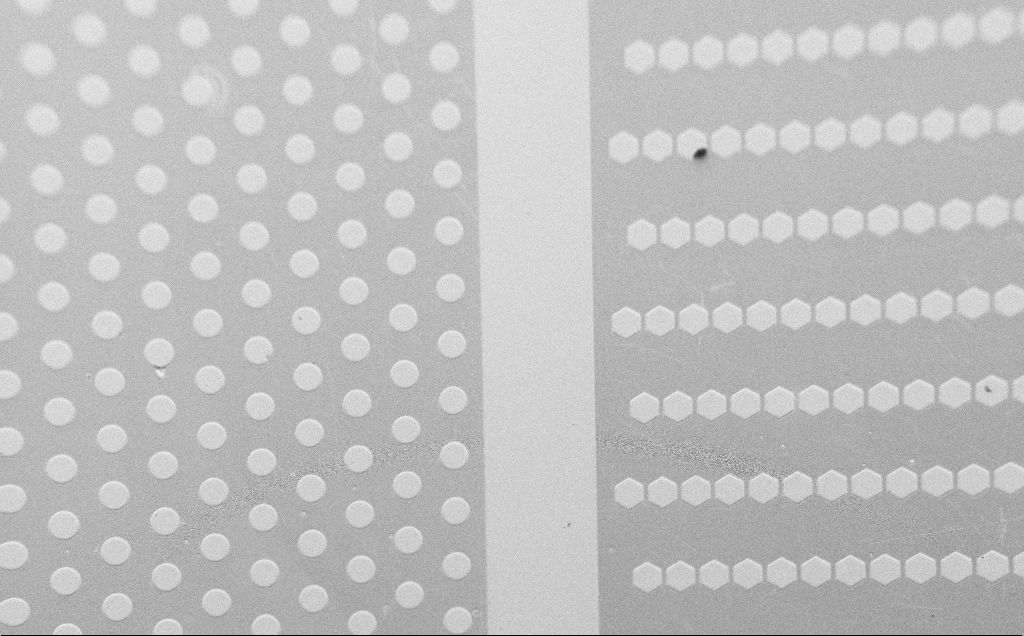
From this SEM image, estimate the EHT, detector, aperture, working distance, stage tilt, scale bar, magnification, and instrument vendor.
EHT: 1.5 kV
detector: SE2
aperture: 30 µm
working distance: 4 mm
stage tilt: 45°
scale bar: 100000 nm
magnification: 0.21 K X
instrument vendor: Zeiss